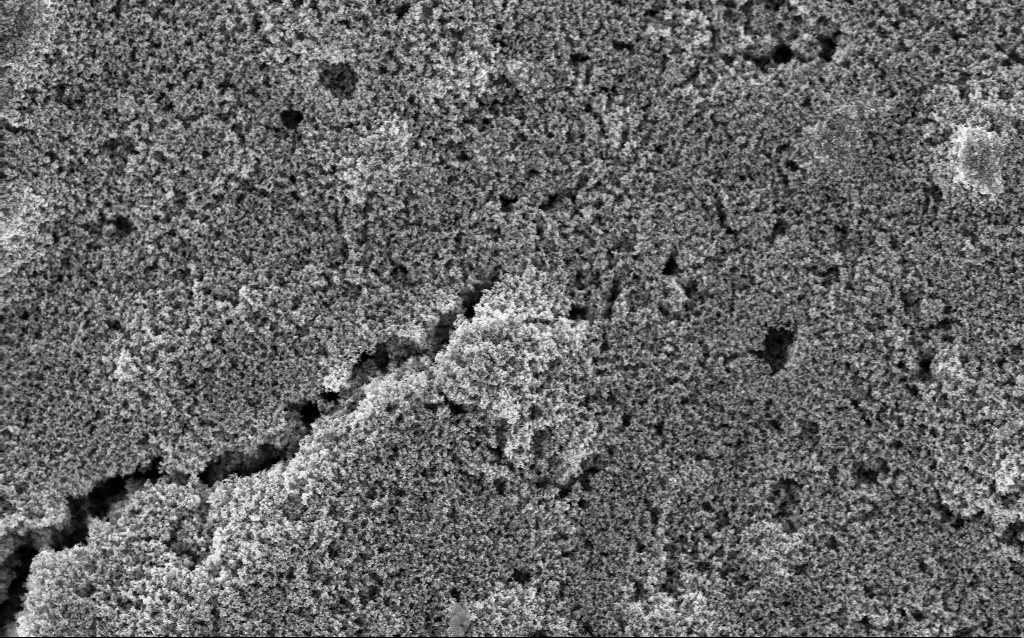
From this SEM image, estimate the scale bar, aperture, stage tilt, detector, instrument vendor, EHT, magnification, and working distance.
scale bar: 1000 nm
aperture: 30 µm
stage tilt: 0°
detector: InLens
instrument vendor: Zeiss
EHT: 5 kV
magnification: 23.9 K X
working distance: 2.9 mm